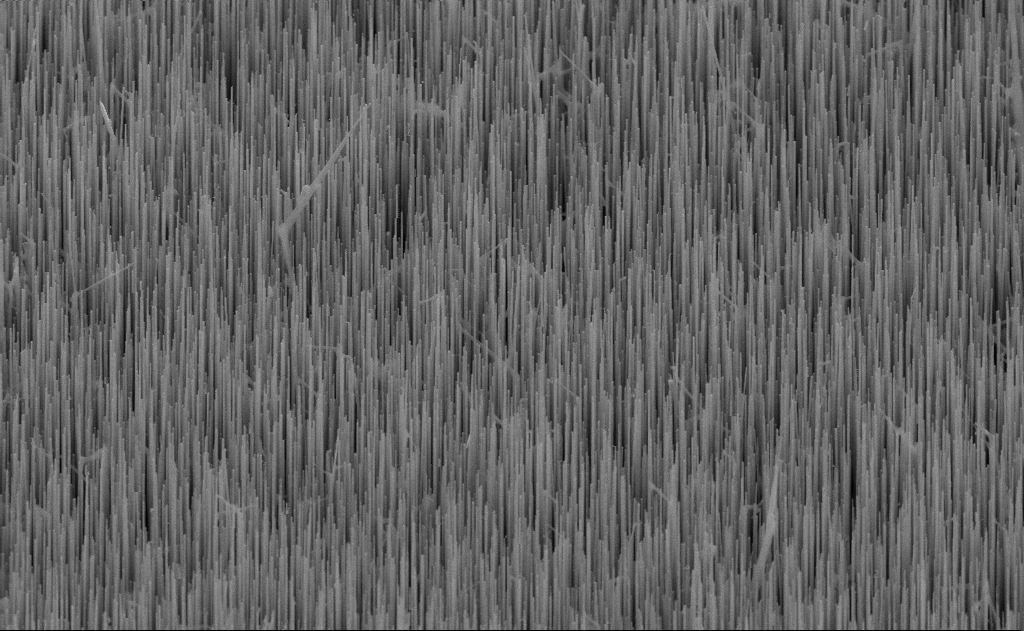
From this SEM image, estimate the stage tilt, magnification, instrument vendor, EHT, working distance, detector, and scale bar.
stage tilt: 45°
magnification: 20 K X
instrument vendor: Zeiss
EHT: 10 kV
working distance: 10 mm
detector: SE2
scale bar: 1000 nm